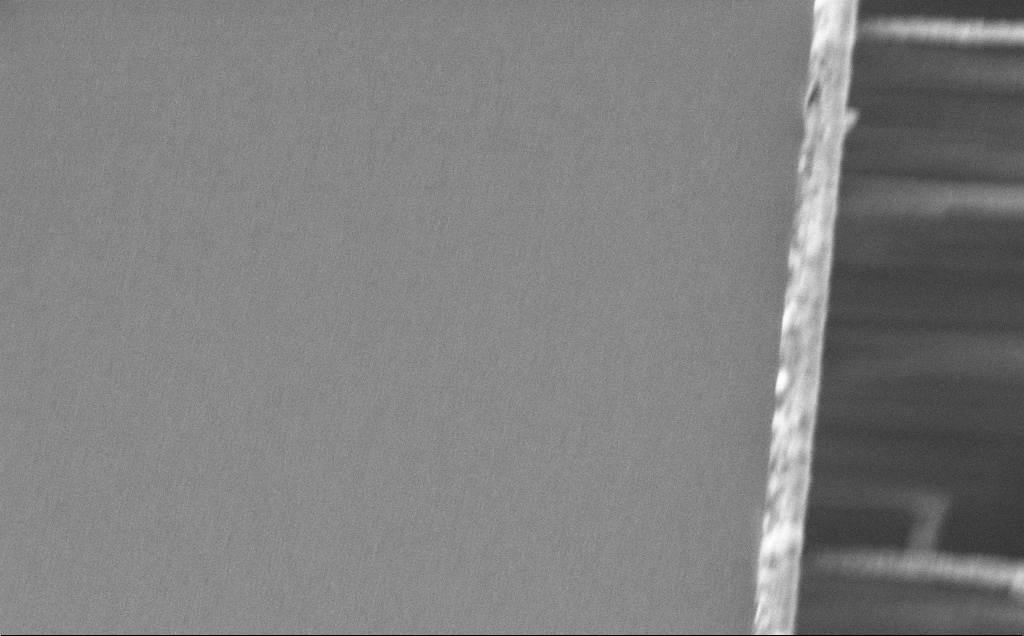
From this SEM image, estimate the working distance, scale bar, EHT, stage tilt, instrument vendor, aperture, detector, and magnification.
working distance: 12 mm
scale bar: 1000 nm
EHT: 5 kV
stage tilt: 0°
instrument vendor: Zeiss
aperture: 30 µm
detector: InLens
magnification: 62.46 K X